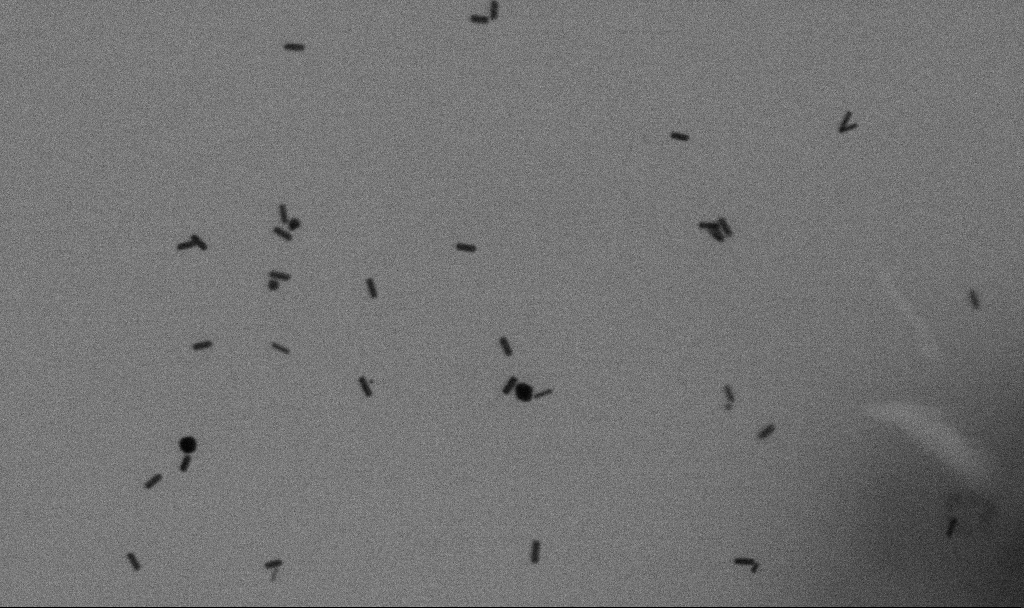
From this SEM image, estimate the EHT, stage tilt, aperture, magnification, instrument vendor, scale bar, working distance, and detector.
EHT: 10 kV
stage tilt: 0°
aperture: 30 µm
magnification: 100 K X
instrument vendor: Zeiss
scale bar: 200 nm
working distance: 4.7 mm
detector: SE2